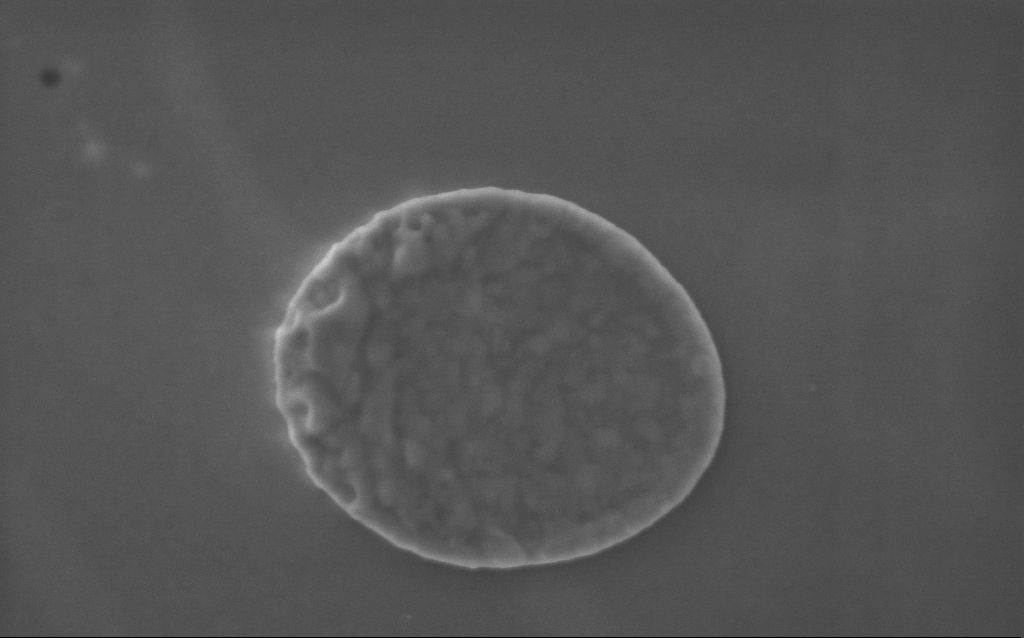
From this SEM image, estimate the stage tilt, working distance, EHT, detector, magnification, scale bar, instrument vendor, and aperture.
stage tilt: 0°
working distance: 3 mm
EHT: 5 kV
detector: InLens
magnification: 91.81 K X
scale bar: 200 nm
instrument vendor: Zeiss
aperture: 30 µm